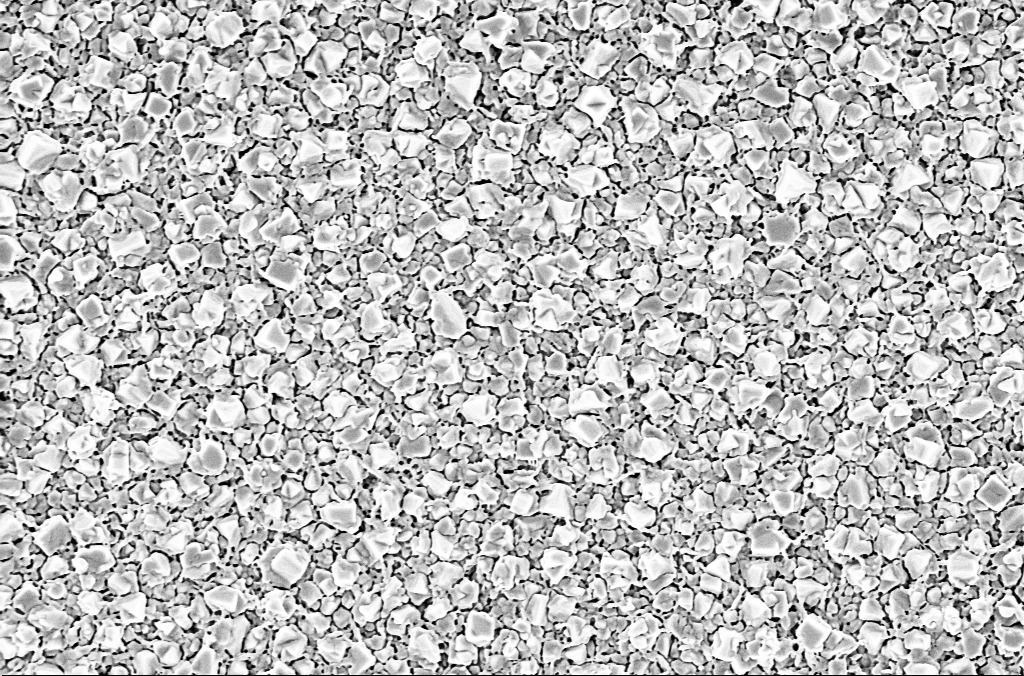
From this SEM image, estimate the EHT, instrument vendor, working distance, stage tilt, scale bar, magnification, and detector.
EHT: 2 kV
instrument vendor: Zeiss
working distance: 1.9 mm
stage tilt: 0°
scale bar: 1000 nm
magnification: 30 K X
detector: InLens